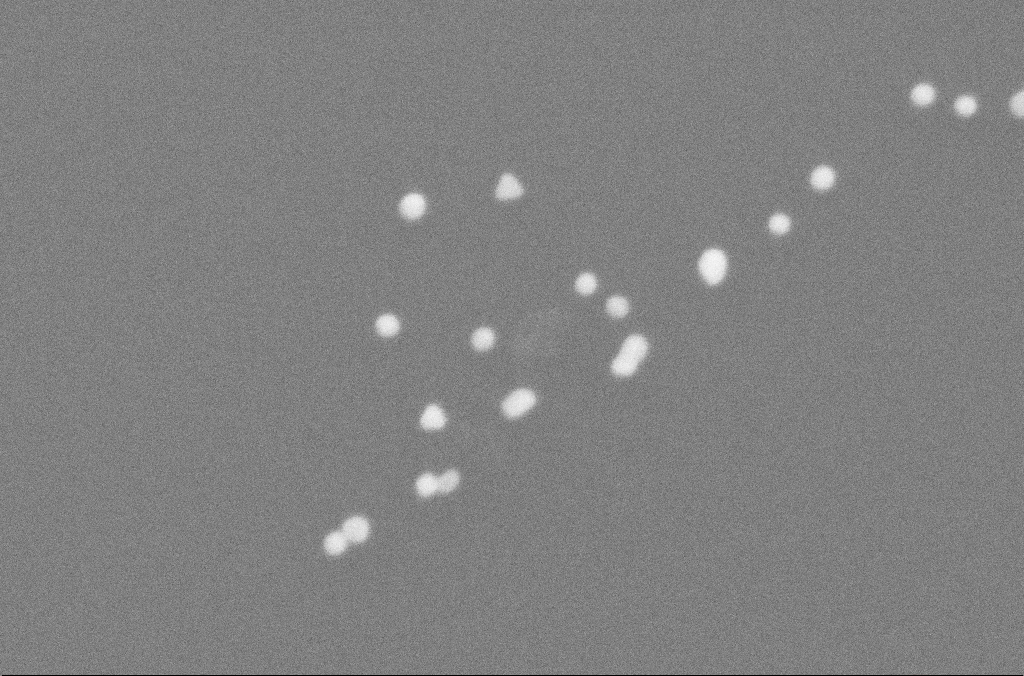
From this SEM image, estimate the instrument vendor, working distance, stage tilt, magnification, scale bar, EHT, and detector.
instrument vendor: Zeiss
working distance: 2.5 mm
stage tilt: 0°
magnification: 435.02 K X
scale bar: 100 nm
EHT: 10 kV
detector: SE2